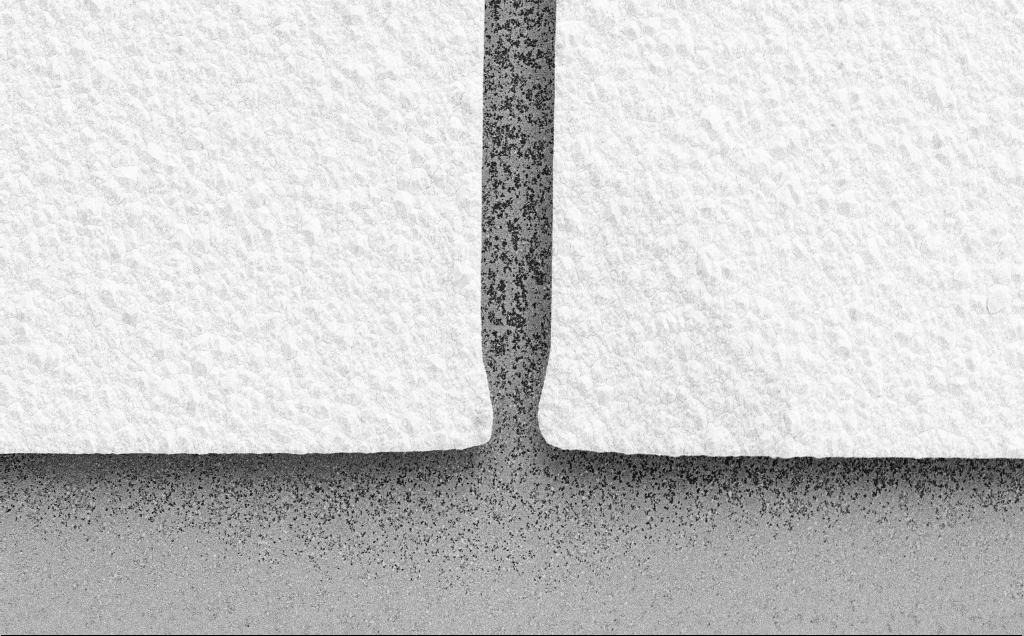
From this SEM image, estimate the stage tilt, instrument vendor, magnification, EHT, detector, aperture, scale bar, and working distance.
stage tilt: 0°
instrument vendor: Zeiss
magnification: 2.77 K X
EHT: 10 kV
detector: SE2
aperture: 30 µm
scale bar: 20000 nm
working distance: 17 mm